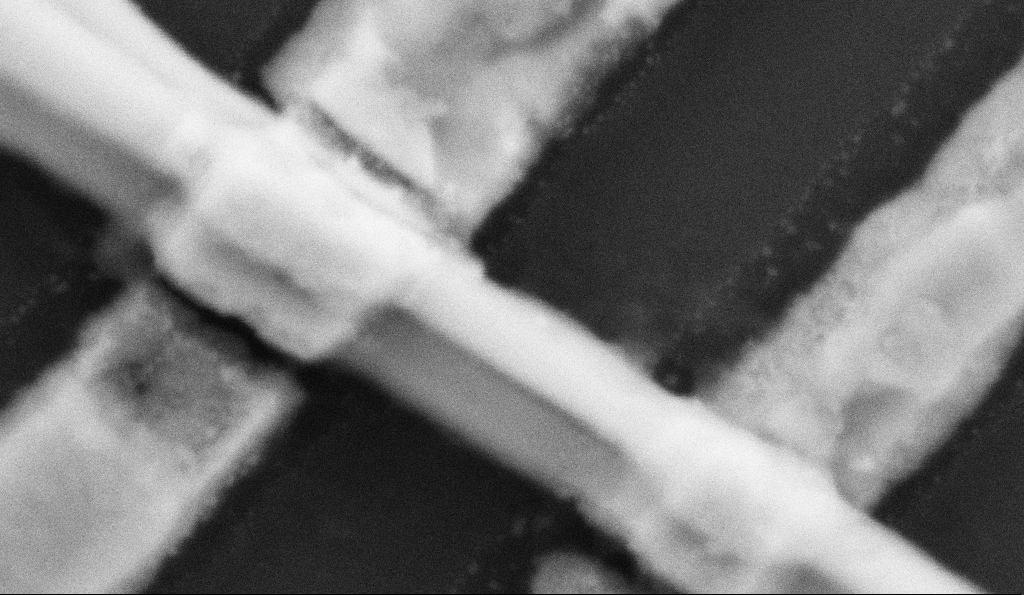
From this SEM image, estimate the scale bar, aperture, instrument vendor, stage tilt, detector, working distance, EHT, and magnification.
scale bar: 100 nm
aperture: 30 µm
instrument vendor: Zeiss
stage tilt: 0°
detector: SE2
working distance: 8.5 mm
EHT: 5 kV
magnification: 300 K X